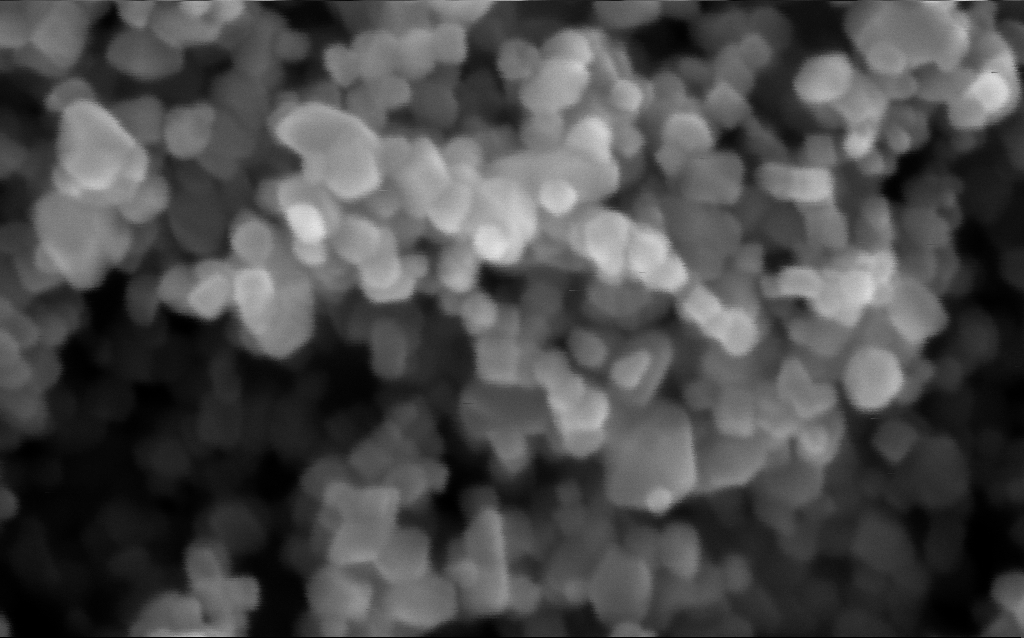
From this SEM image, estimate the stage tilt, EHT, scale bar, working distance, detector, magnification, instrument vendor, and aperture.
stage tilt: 0°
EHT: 5 kV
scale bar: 100 nm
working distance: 5.3 mm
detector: InLens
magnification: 716 K X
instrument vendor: Zeiss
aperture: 30 µm